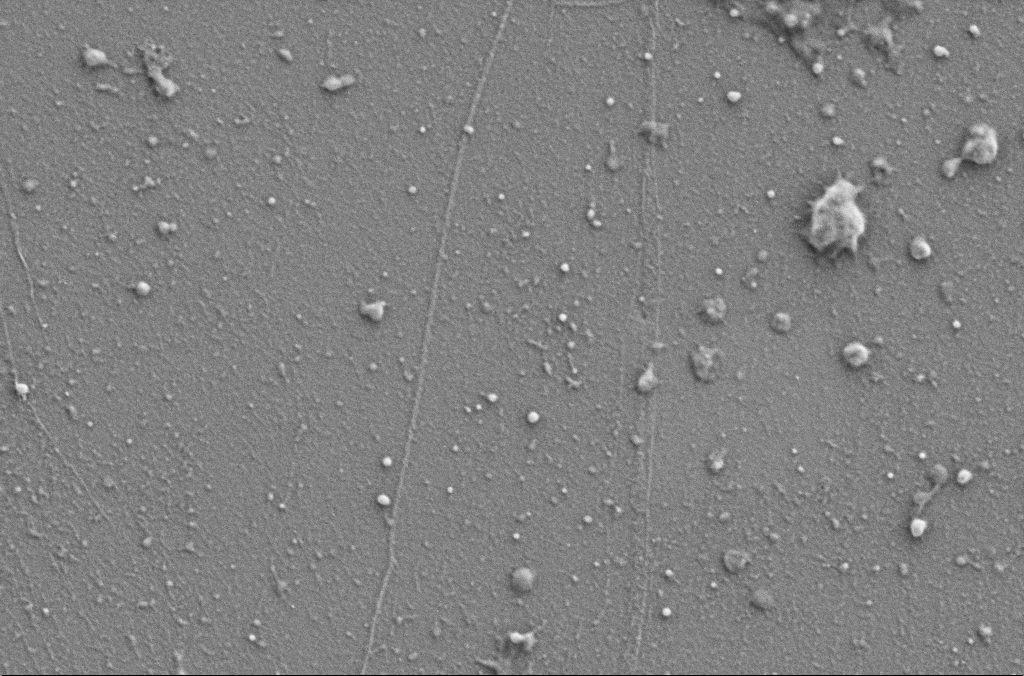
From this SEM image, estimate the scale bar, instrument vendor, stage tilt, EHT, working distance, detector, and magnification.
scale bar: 1000 nm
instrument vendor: Zeiss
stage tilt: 0°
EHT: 1 kV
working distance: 4.1 mm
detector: SE2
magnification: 50 K X